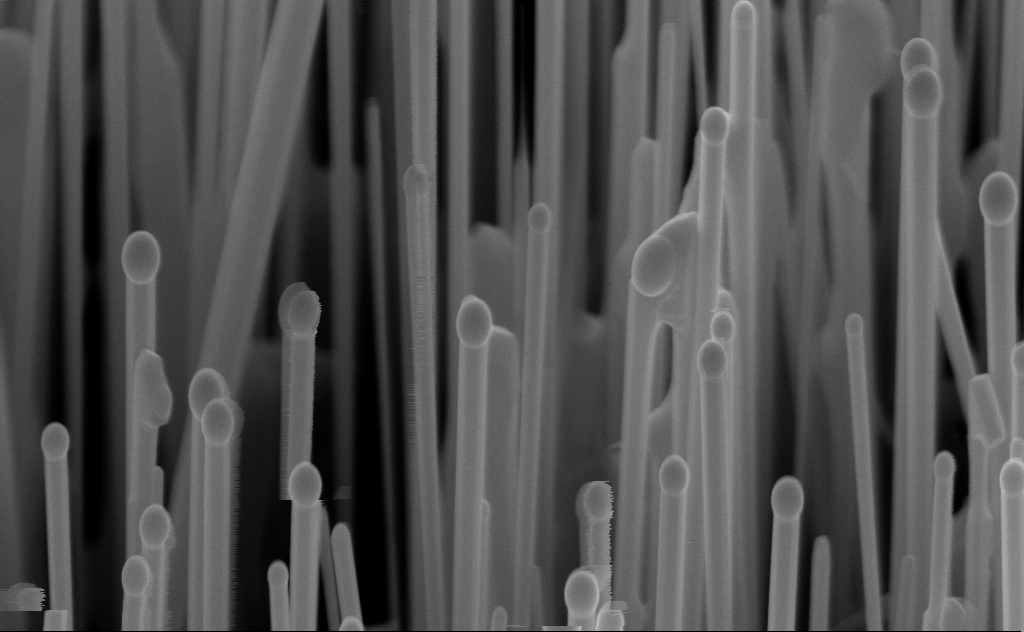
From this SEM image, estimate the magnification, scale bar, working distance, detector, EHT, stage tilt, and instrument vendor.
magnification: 80 K X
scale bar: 200 nm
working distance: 6 mm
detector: InLens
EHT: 10 kV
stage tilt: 45°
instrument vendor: Zeiss